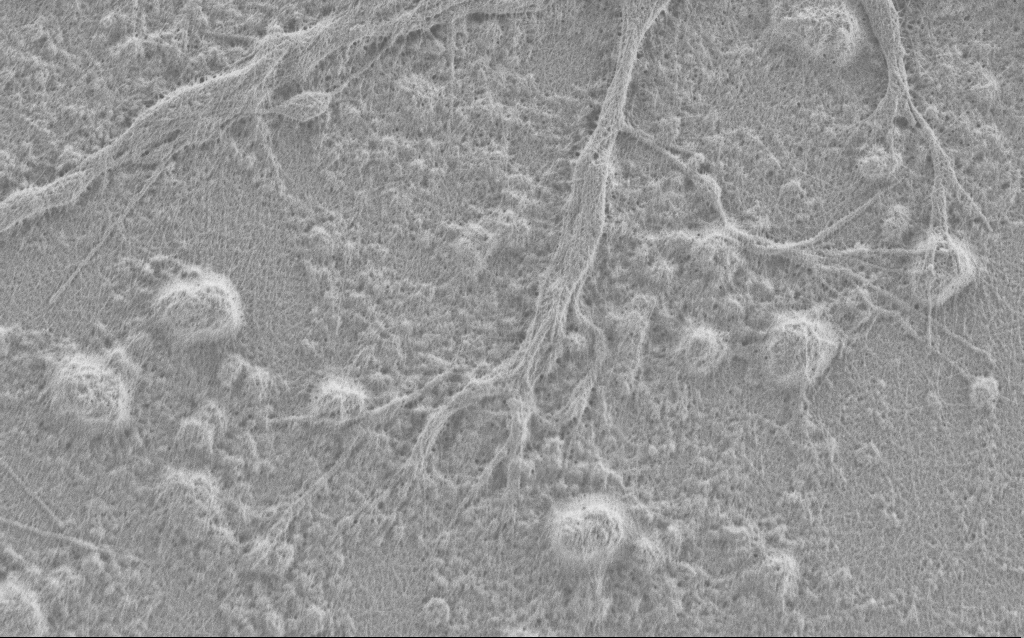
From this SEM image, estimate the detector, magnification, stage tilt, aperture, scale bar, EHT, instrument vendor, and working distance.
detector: SE2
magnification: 10 K X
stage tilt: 0°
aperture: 30 µm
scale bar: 2000 nm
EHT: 0.9 kV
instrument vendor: Zeiss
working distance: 4 mm